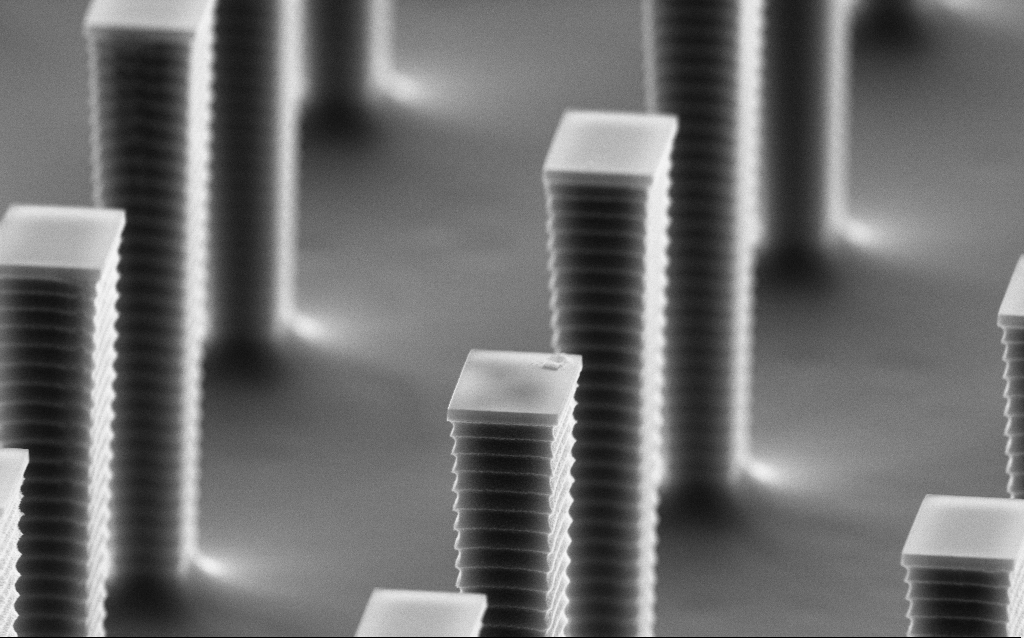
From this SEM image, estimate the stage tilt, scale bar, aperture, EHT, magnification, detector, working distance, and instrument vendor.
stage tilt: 70°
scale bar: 1000 nm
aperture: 30 µm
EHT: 8 kV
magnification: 20.58 K X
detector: SE2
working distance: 8.5 mm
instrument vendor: Zeiss